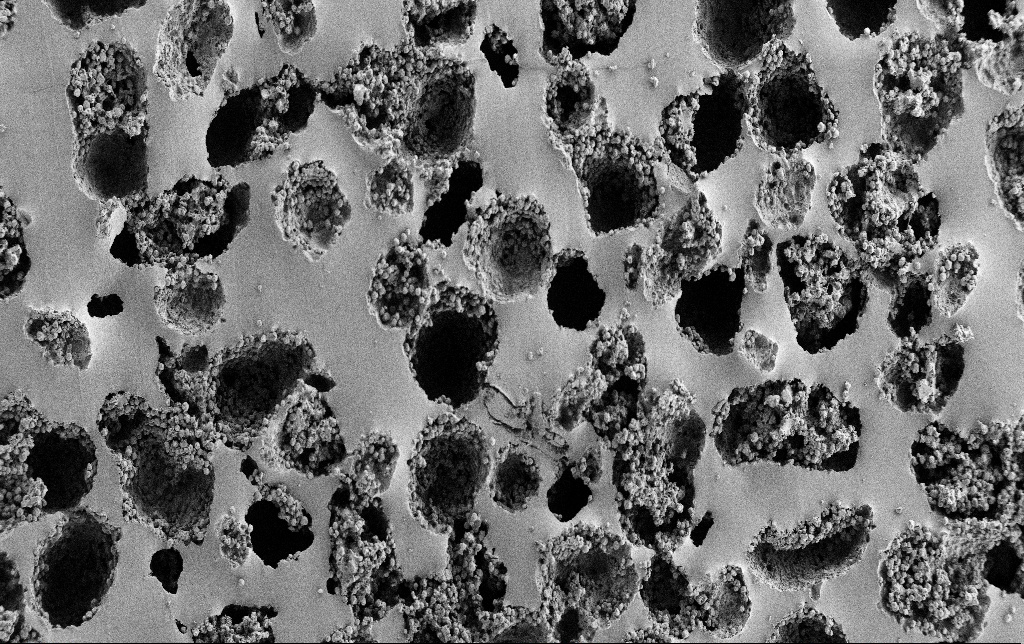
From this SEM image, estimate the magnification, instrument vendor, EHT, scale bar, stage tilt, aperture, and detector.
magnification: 0.15 K X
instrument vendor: Zeiss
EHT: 3 kV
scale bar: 100000 nm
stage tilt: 0°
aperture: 30 µm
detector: SE2